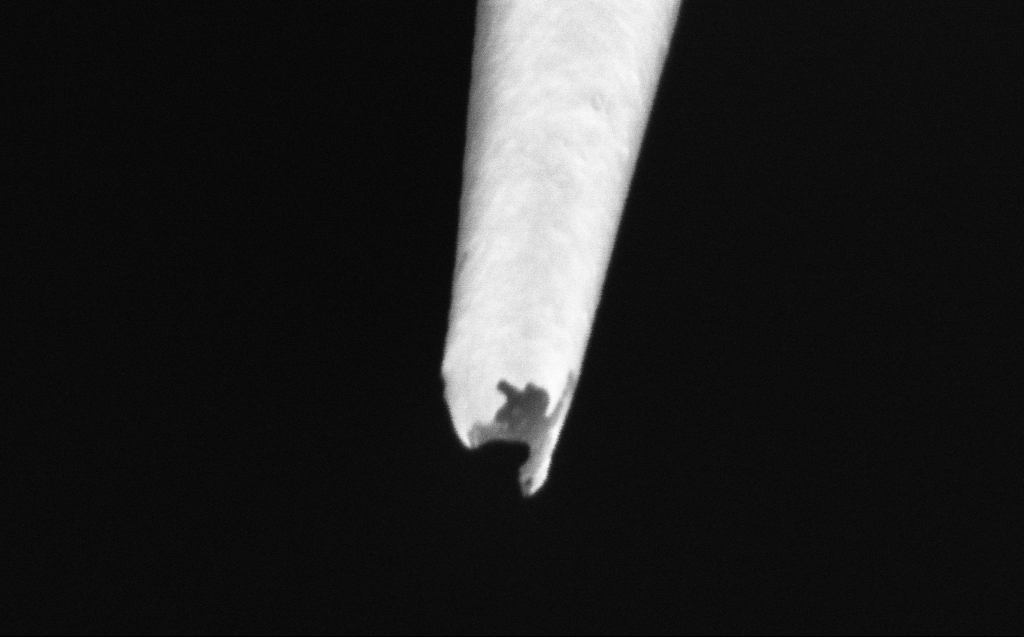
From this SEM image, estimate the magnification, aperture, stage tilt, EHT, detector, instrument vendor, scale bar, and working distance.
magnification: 250 K X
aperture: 30 µm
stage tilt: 45°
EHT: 2 kV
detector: InLens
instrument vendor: Zeiss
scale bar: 200 nm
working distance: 4 mm